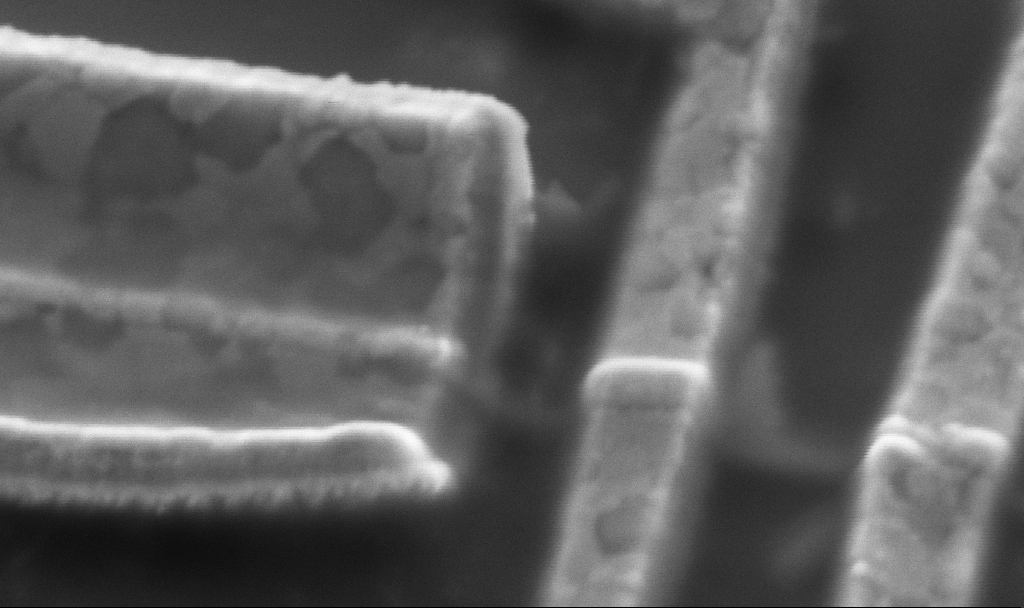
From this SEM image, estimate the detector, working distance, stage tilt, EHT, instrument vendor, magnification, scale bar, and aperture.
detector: SE2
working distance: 16.7 mm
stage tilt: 45°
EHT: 5 kV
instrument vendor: Zeiss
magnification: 100 K X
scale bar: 200 nm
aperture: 30 µm